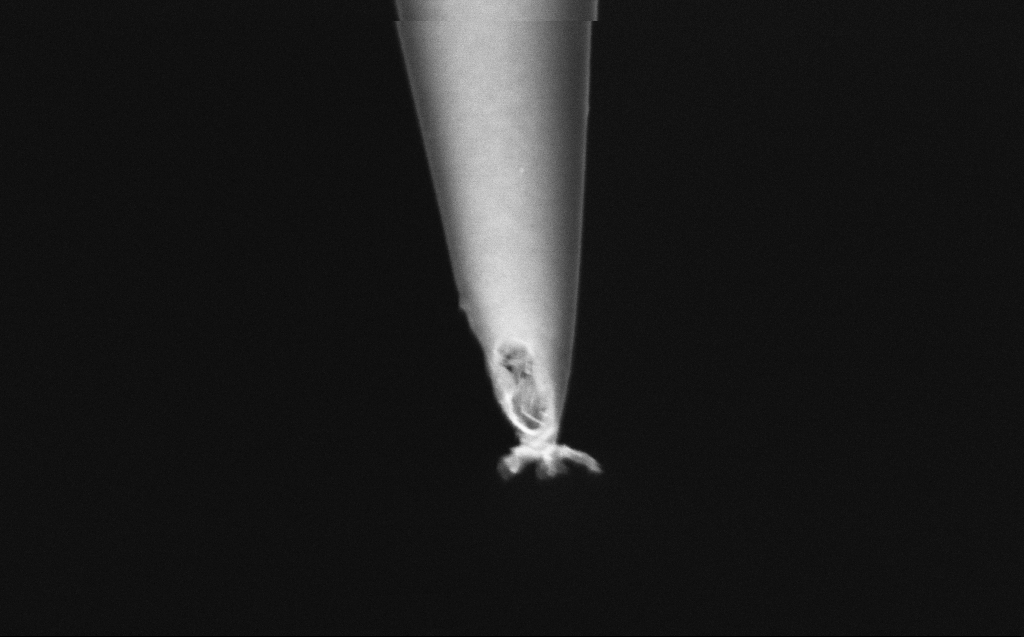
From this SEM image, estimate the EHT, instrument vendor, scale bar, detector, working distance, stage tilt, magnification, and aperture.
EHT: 2 kV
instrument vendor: Zeiss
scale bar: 200 nm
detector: InLens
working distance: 6 mm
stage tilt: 45°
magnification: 100 K X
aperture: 30 µm